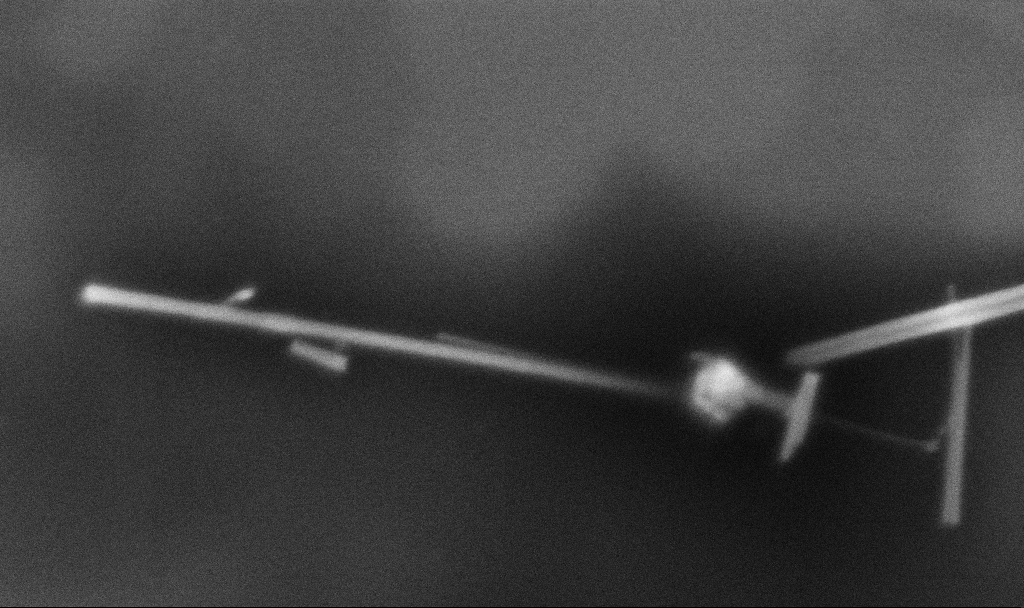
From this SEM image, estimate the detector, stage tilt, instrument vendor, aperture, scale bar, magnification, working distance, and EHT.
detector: InLens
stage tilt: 0°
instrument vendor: Zeiss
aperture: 30 µm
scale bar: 100 nm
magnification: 163.71 K X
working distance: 3.3 mm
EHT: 3 kV